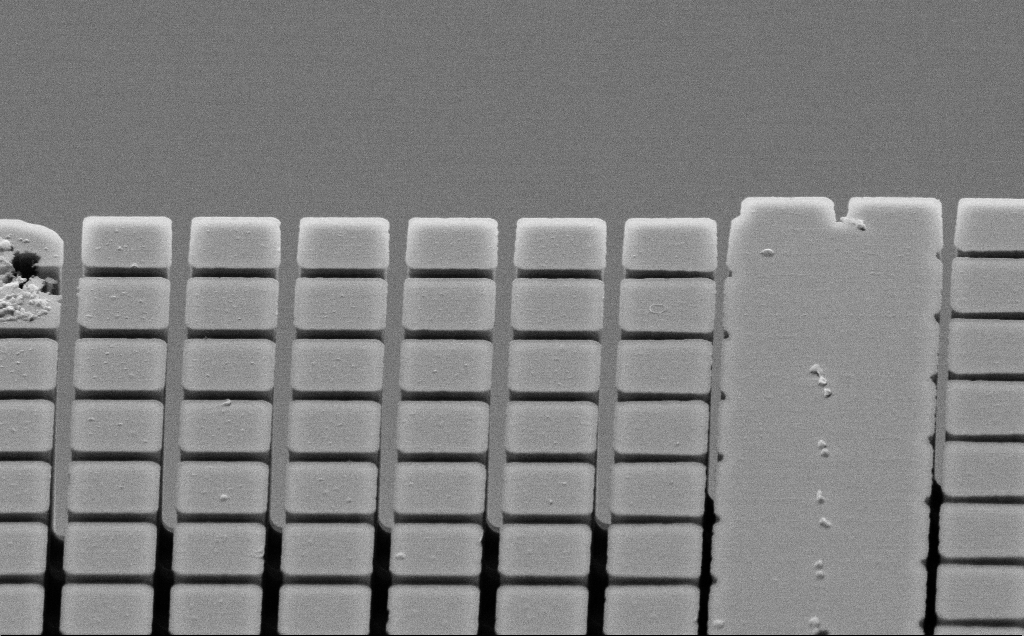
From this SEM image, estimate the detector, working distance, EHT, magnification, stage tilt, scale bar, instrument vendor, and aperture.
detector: SE2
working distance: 8 mm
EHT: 10 kV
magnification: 8.05 K X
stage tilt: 60.9°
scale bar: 2000 nm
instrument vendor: Zeiss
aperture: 30 µm